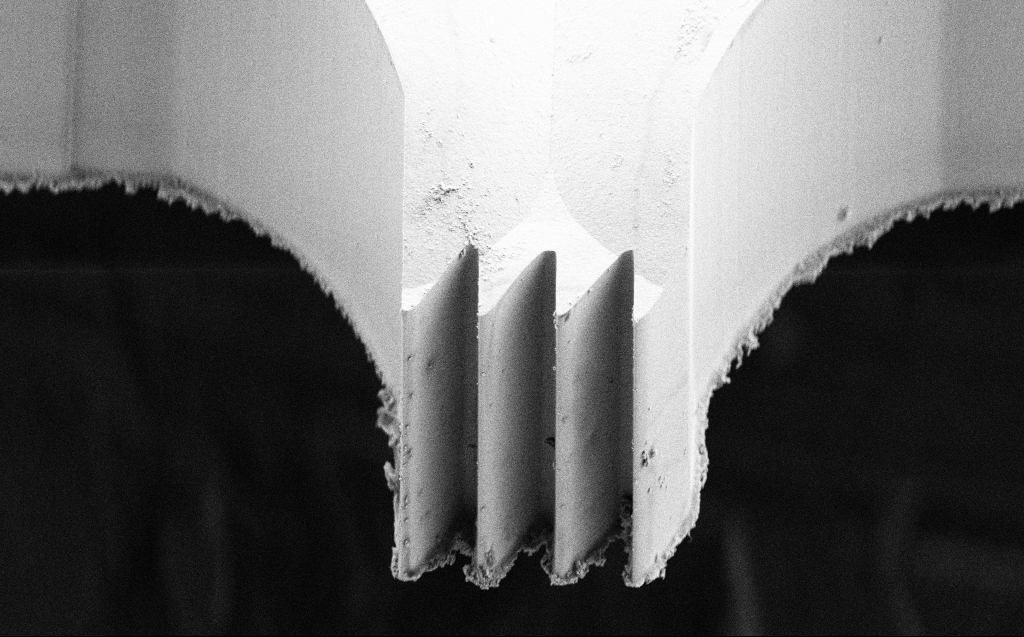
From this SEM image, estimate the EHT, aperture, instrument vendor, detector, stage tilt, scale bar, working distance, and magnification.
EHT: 5 kV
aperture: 30 µm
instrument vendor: Zeiss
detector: SE2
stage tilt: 45°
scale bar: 10000 nm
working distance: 5 mm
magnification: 2.54 K X